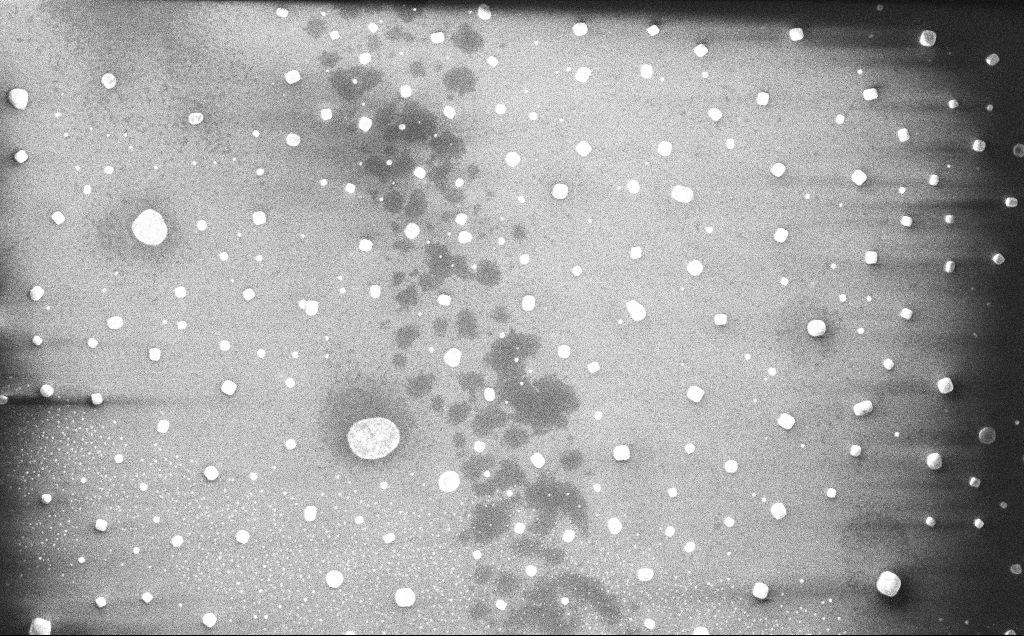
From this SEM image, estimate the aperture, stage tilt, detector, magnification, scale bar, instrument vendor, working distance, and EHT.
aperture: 30 µm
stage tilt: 0°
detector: InLens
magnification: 16.36 K X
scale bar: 2000 nm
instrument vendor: Zeiss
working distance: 5 mm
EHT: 10 kV